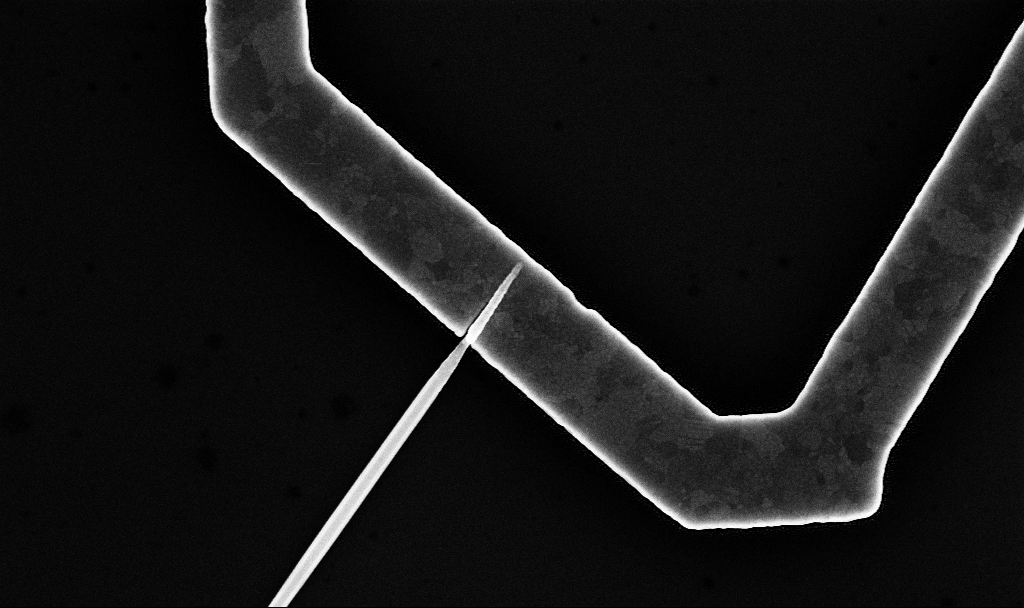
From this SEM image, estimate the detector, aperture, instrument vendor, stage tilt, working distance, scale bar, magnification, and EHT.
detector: InLens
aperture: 30 µm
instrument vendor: Zeiss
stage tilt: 0°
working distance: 7.7 mm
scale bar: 1000 nm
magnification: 43.45 K X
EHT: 10 kV